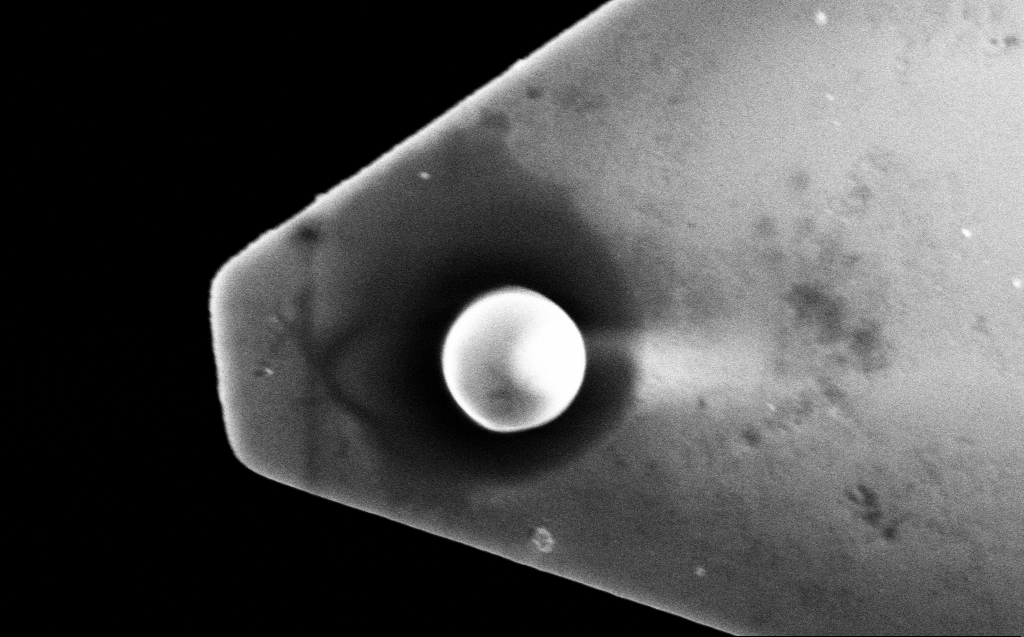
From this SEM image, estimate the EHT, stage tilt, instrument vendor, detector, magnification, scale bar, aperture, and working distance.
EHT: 10 kV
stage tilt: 0°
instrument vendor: Zeiss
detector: SE2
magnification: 11.64 K X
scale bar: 2000 nm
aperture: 30 µm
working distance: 19 mm